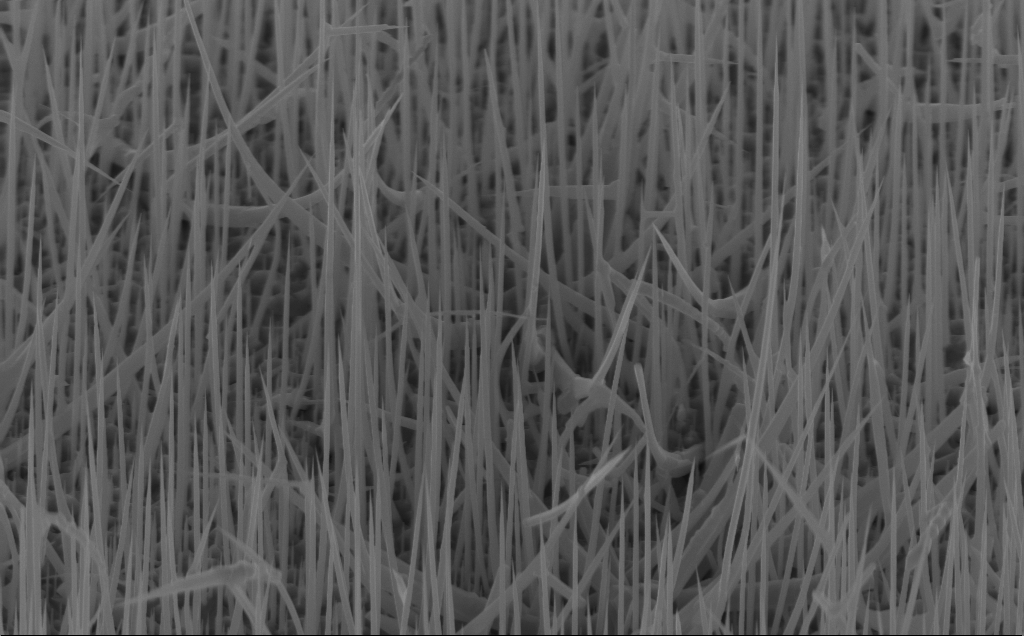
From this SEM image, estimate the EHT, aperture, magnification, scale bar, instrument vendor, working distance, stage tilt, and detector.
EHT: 10 kV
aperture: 30 µm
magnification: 20 K X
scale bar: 1000 nm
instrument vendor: Zeiss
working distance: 7 mm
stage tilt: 45°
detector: InLens